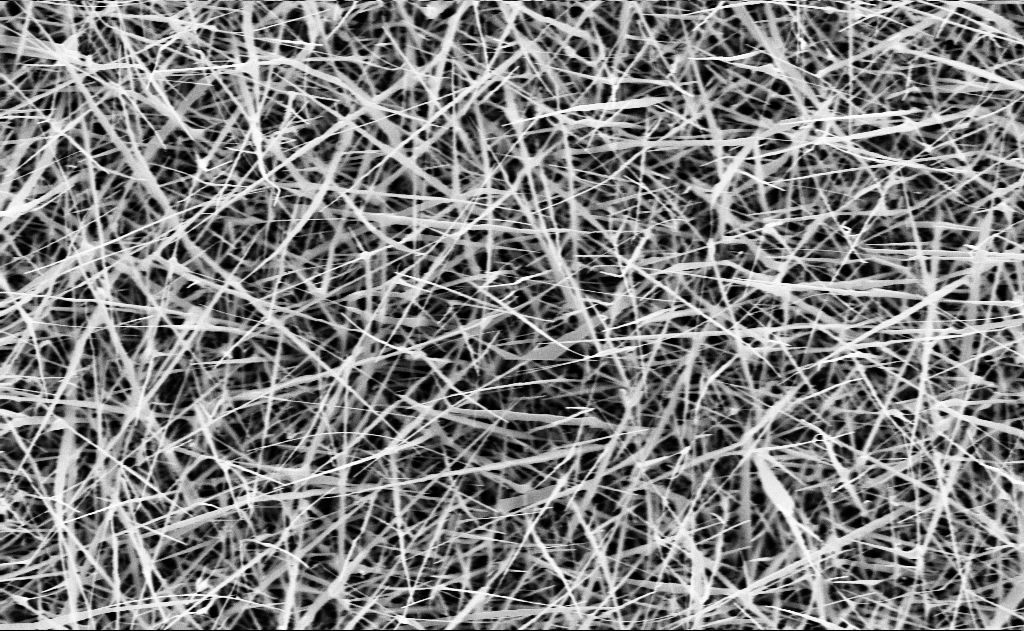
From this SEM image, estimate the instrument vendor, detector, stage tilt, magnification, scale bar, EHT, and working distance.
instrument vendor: Zeiss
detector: InLens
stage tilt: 0°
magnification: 20 K X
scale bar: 1000 nm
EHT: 10 kV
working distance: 15 mm